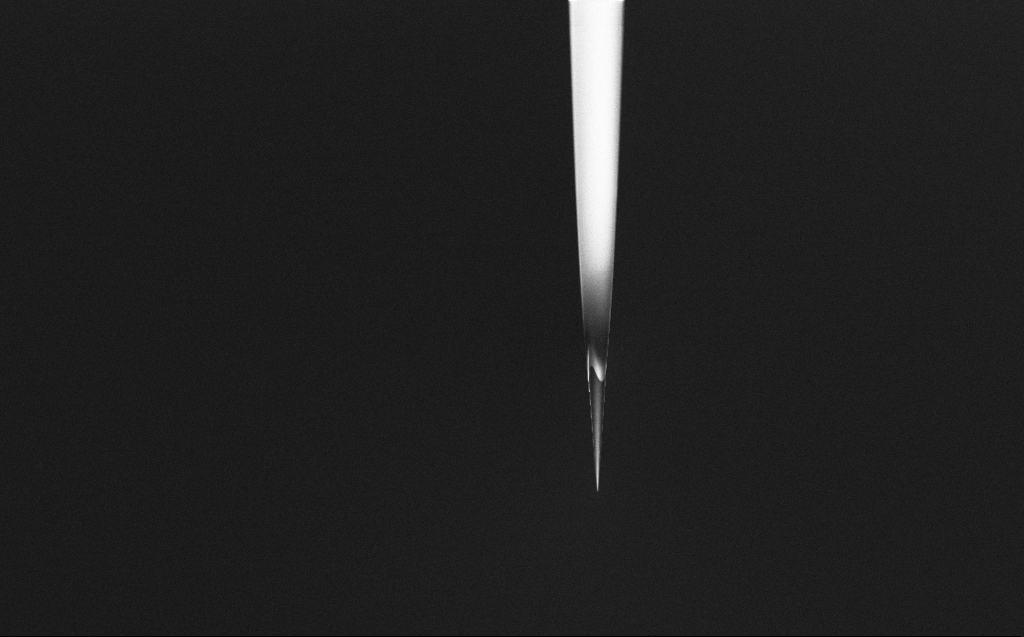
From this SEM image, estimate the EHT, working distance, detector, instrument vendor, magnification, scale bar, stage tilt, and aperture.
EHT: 2 kV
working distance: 6 mm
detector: InLens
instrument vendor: Zeiss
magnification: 1 K X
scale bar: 20000 nm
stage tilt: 45°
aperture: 30 µm